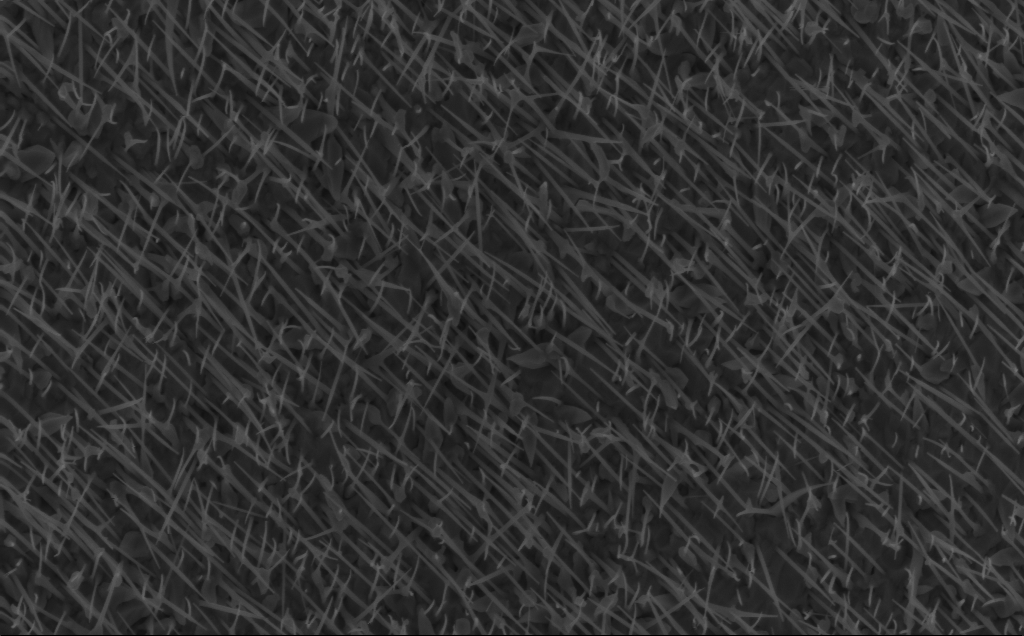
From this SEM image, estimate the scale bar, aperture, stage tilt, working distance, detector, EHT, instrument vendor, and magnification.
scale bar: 1000 nm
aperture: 30 µm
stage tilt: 0°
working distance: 5 mm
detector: InLens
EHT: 5 kV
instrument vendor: Zeiss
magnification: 20 K X